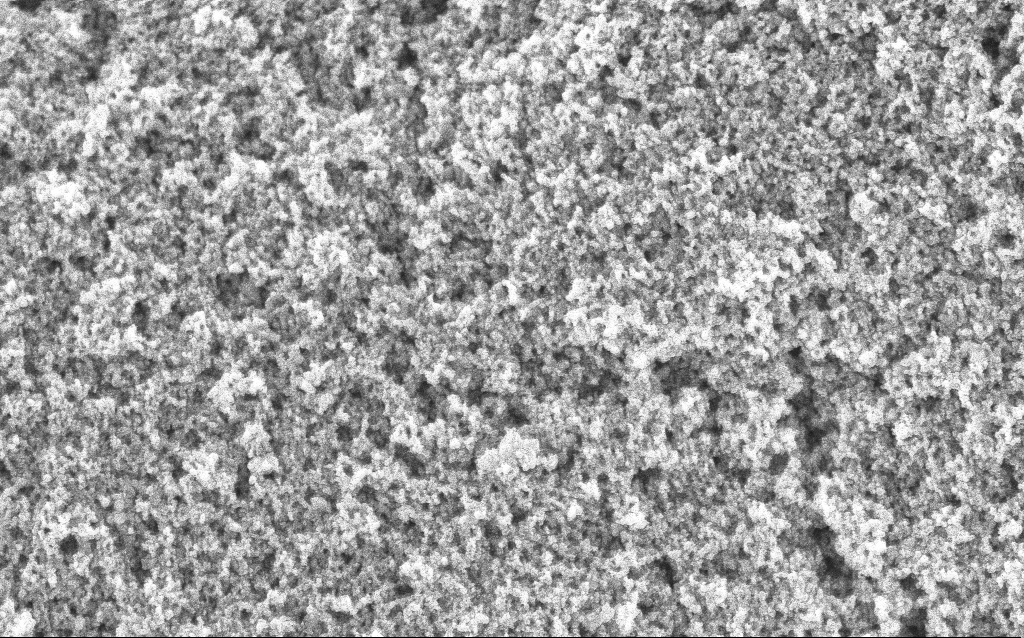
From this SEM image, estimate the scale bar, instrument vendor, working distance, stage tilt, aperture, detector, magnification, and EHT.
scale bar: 1000 nm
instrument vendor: Zeiss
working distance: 4.4 mm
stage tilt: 0°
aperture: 30 µm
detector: InLens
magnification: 65.04 K X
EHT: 5 kV